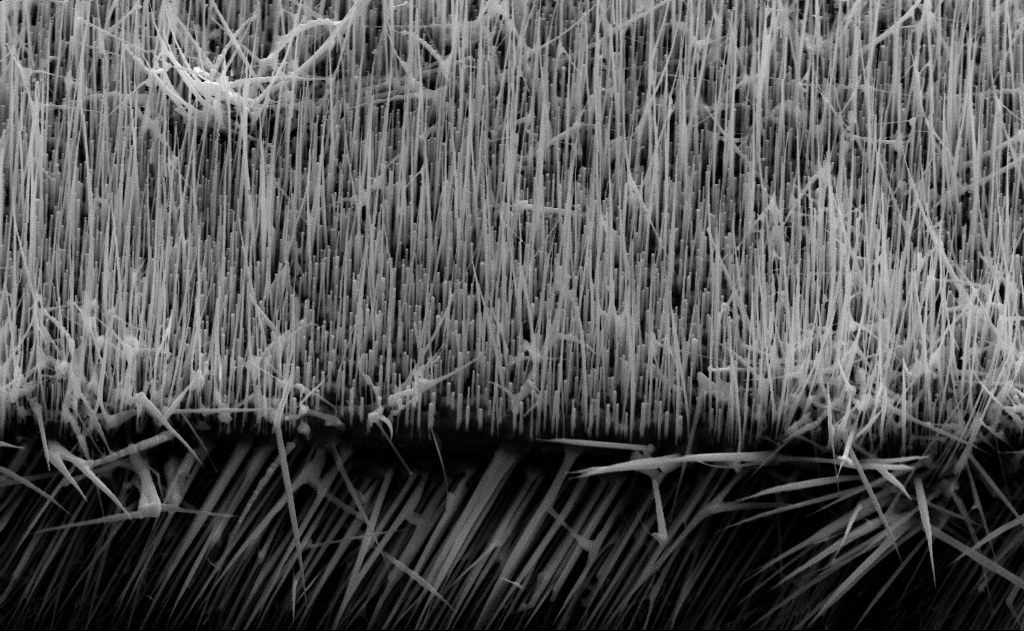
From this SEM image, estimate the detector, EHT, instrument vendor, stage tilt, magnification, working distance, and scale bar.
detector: SE2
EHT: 10 kV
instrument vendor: Zeiss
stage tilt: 45°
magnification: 20 K X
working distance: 16 mm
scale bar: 1000 nm